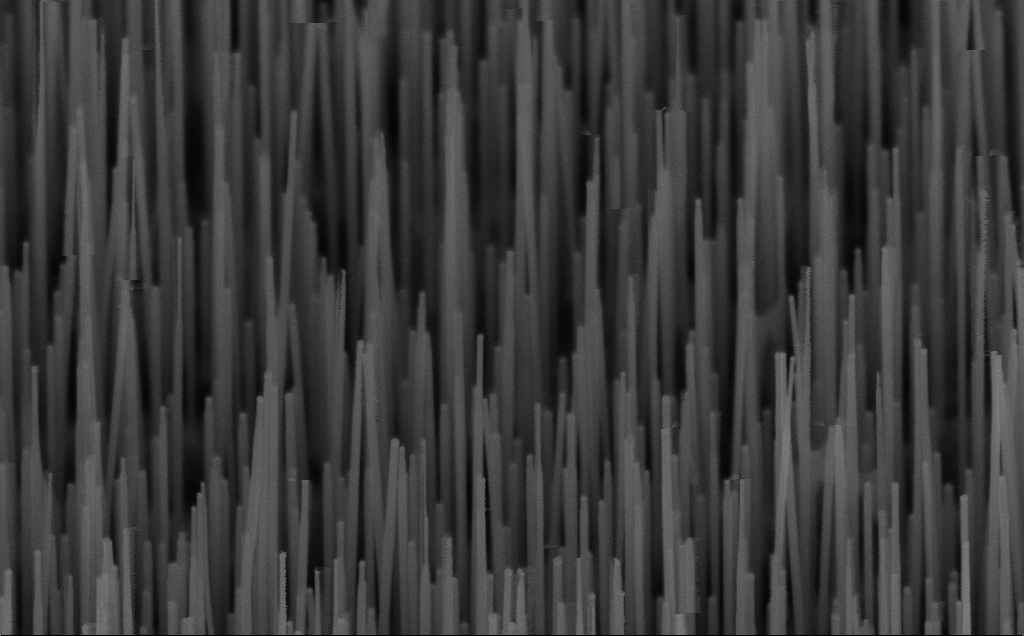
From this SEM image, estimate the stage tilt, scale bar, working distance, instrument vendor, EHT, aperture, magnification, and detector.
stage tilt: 45°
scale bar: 200 nm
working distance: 7 mm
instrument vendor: Zeiss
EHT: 10 kV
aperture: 30 µm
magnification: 100 K X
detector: InLens